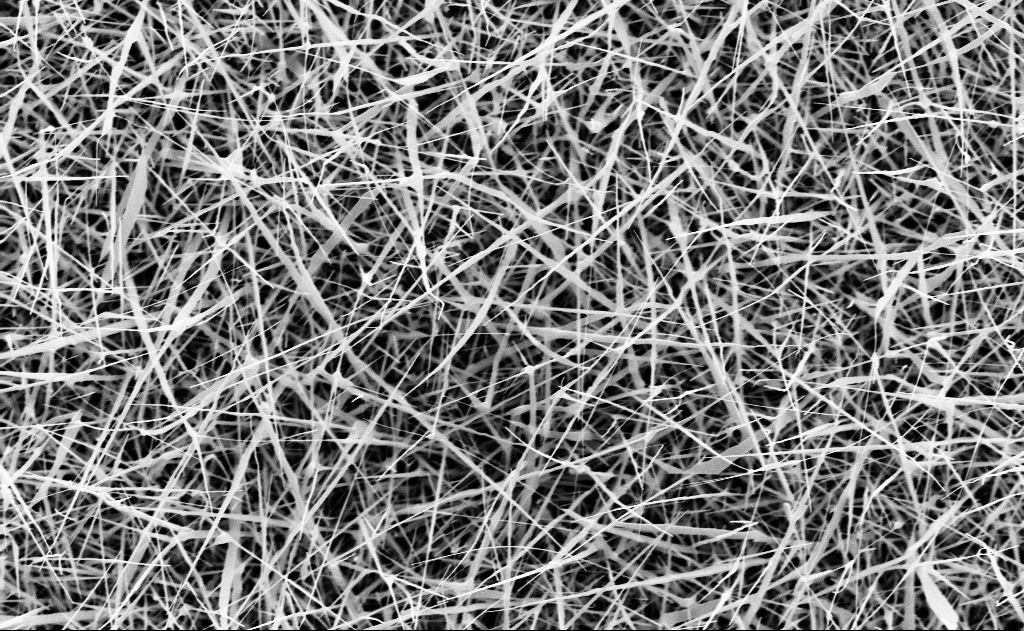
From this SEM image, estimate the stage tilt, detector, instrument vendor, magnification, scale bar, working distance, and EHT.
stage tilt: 0°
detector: InLens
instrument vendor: Zeiss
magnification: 20 K X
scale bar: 1000 nm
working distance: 15 mm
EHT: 10 kV